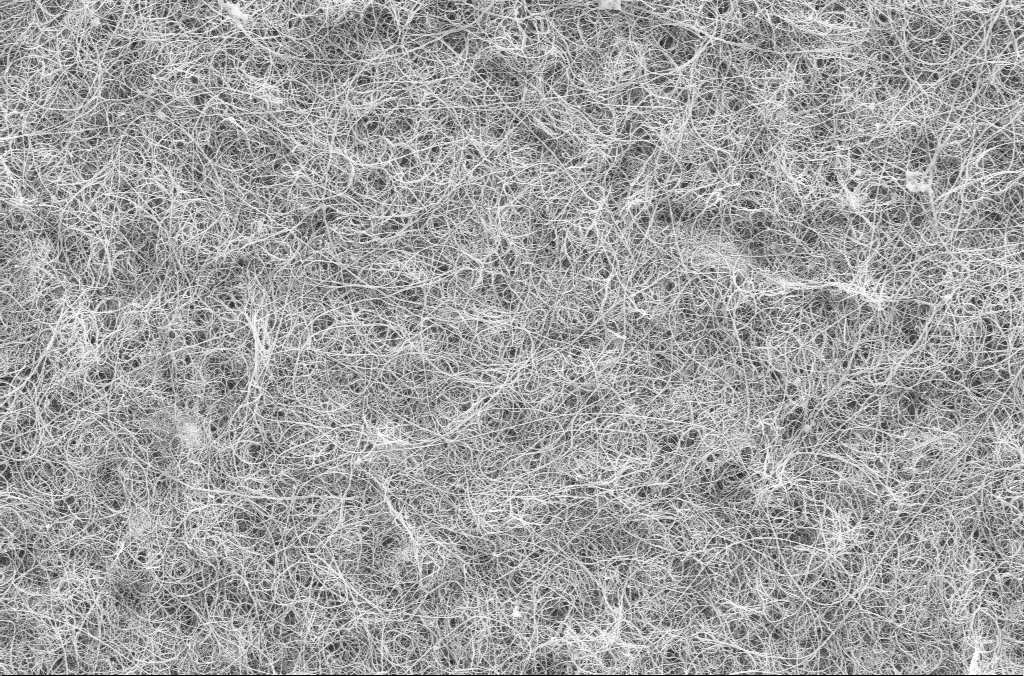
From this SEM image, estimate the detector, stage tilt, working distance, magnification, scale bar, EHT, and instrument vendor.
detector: SE2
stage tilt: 0°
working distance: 3 mm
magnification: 10 K X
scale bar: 2000 nm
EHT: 5 kV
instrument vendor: Zeiss